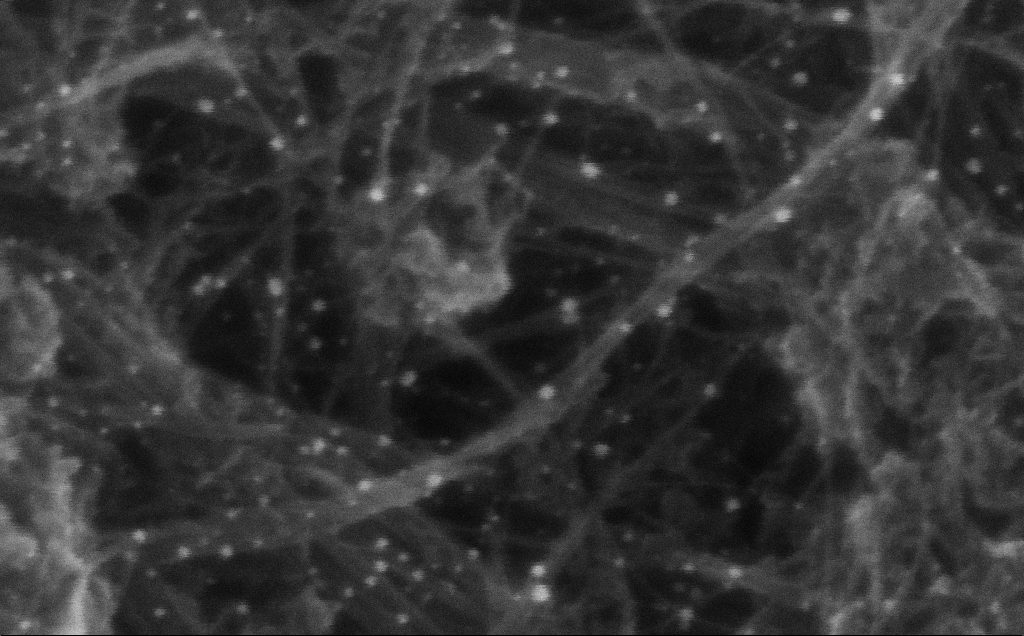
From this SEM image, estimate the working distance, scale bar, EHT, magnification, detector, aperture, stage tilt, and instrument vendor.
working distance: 3 mm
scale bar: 100 nm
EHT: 10 kV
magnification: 515.53 K X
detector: InLens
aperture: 30 µm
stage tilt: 0°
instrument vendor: Zeiss